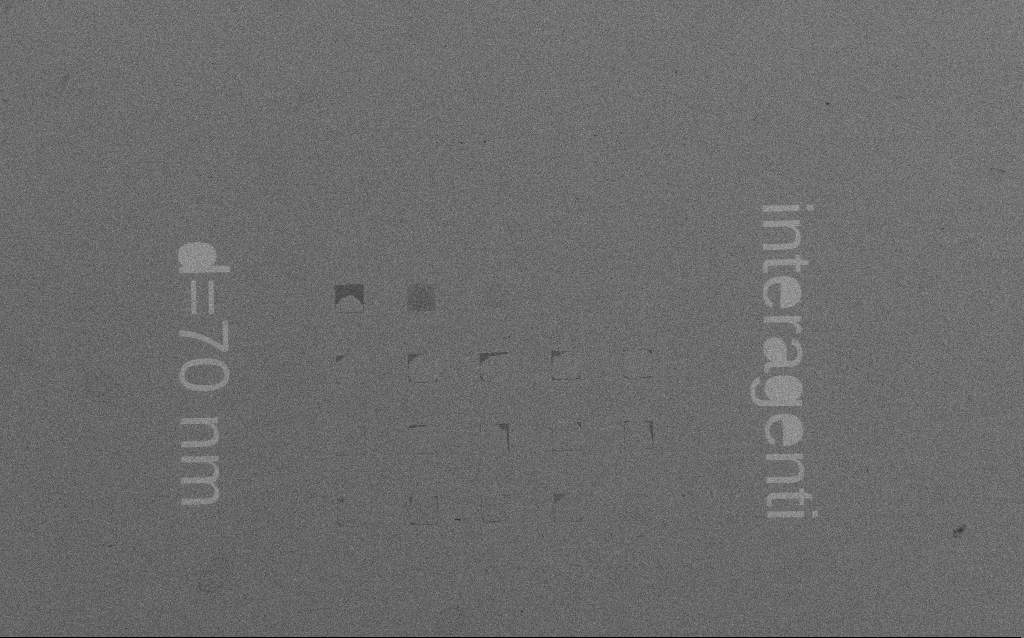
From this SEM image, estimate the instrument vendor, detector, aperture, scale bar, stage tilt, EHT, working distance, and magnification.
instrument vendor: Zeiss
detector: SE2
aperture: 30 µm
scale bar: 200000 nm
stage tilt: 0°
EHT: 1.5 kV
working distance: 5.8 mm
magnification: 0.35 K X